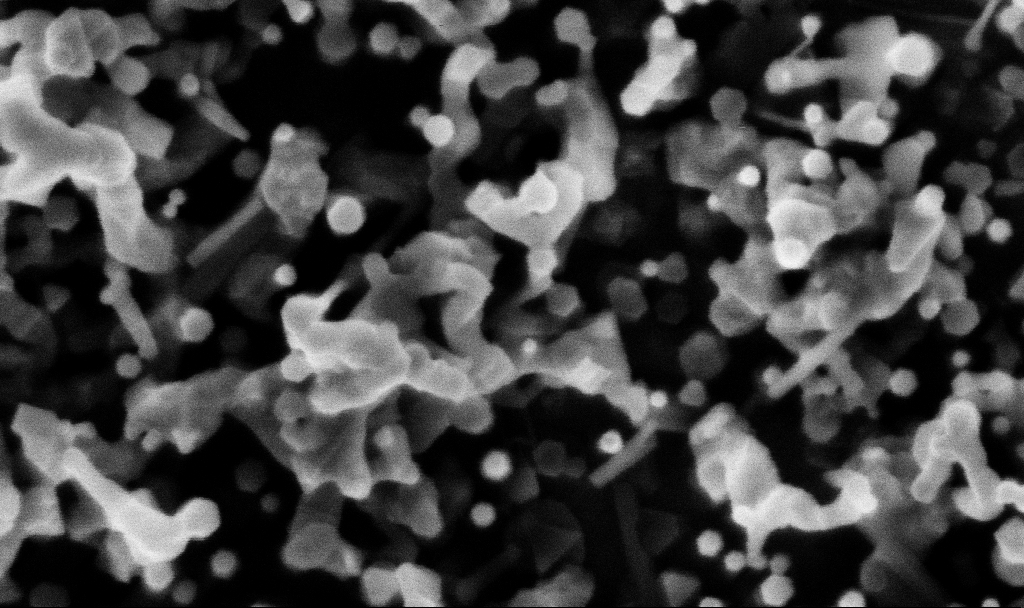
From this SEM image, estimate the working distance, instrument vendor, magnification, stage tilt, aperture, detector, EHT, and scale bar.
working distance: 3.1 mm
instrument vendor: Zeiss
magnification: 254.66 K X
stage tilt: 0°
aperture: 30 µm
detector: InLens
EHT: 3 kV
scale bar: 200 nm